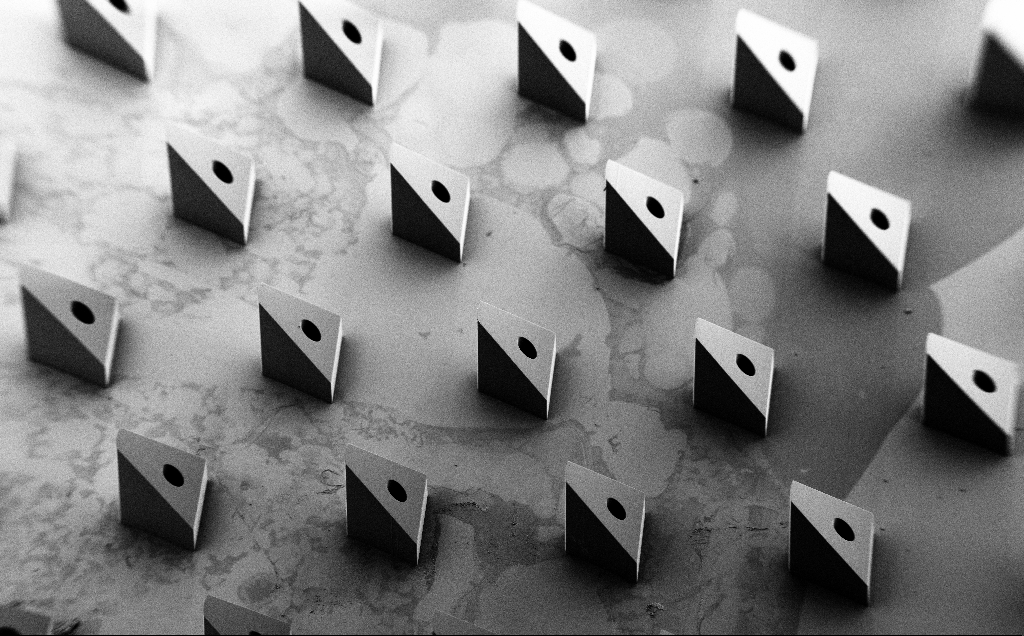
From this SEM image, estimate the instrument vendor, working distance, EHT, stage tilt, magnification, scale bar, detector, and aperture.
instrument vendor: Zeiss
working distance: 8 mm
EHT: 5 kV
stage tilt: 35°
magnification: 0.082 K X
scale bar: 200000 nm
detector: SE2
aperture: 30 µm